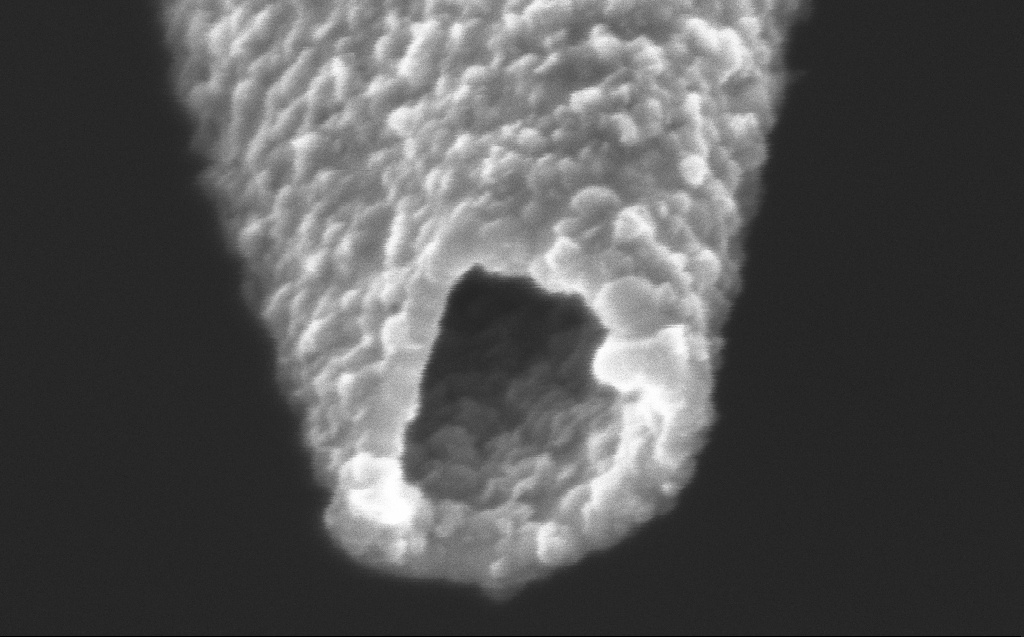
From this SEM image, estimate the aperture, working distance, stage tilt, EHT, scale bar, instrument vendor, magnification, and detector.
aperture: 30 µm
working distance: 3 mm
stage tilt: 45°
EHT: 2 kV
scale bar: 200 nm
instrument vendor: Zeiss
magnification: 285.88 K X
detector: InLens